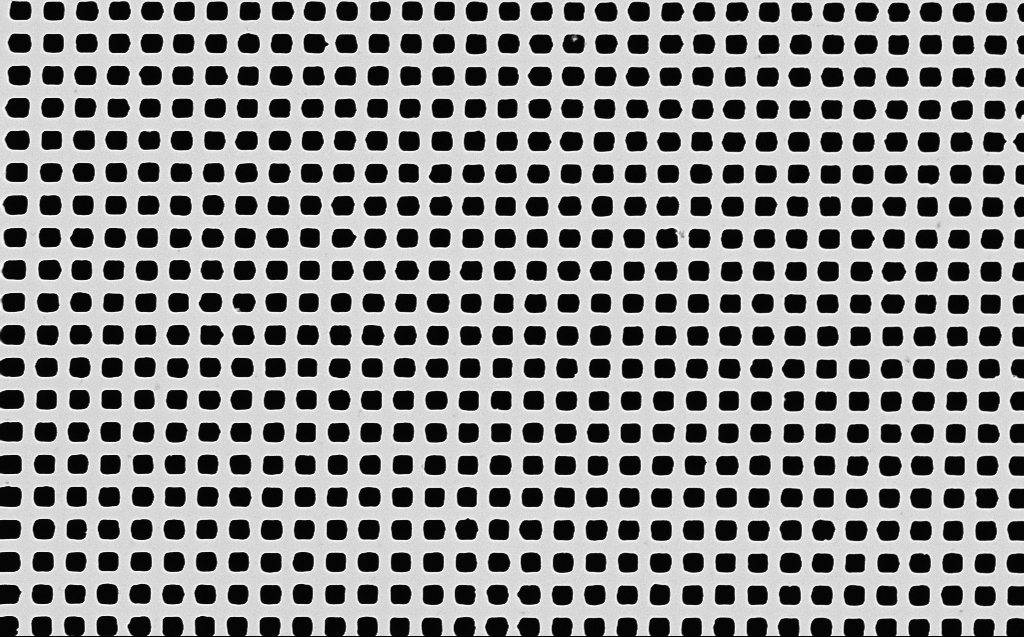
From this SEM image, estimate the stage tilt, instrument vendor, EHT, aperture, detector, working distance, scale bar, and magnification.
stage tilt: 0°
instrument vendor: Zeiss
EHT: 10 kV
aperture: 30 µm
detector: InLens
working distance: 7 mm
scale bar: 1000 nm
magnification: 24.08 K X